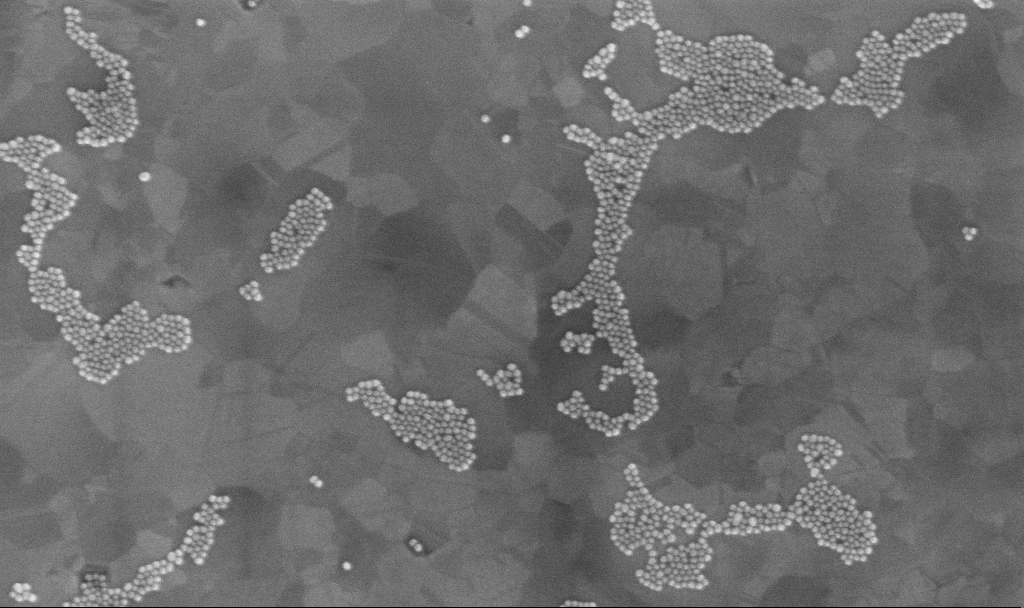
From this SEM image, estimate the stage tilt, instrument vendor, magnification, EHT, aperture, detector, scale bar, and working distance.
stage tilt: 0°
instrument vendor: Zeiss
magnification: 150 K X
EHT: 10 kV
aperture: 30 µm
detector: InLens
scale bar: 100 nm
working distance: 3.4 mm